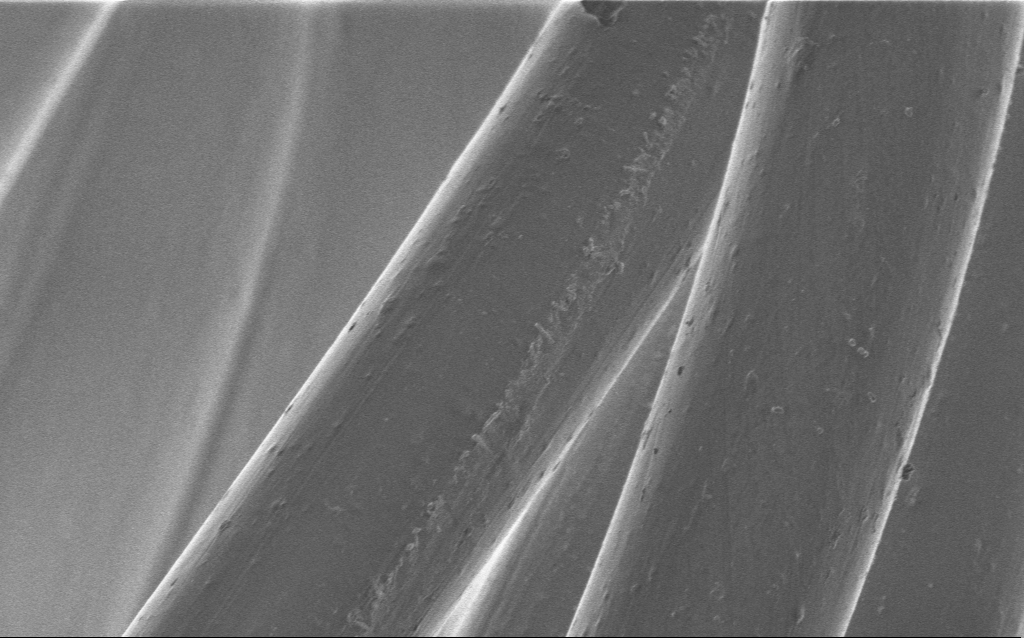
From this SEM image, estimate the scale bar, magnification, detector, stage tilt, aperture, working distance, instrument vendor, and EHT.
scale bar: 10000 nm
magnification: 3.95 K X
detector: InLens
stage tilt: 0°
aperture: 30 µm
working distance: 4 mm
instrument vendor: Zeiss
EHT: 1 kV